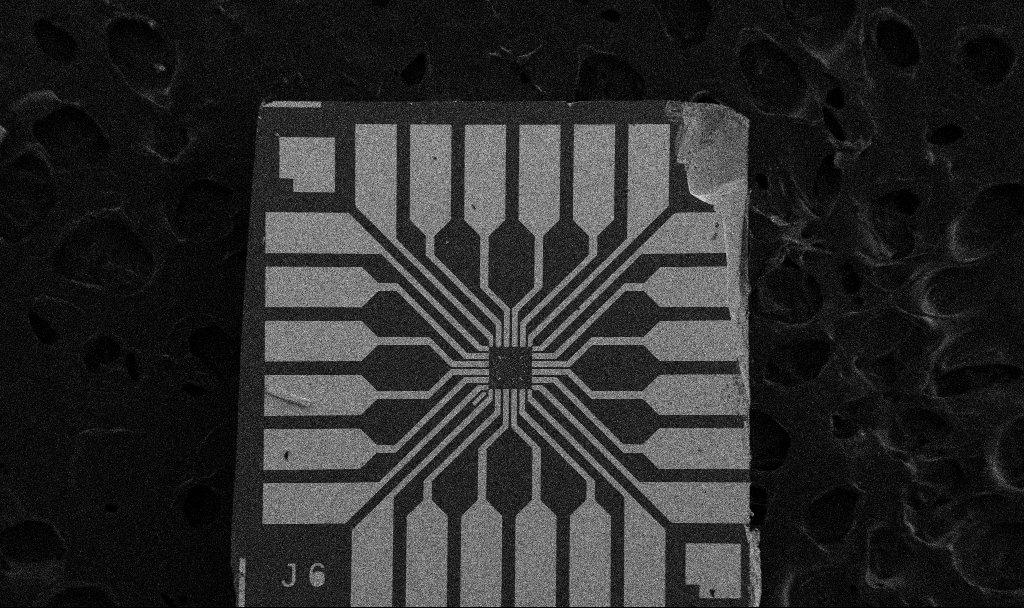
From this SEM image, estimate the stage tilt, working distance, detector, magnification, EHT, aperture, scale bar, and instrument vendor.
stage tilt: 0°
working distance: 10.7 mm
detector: SE2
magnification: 0.1 K X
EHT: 5 kV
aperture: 30 µm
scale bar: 200000 nm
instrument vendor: Zeiss